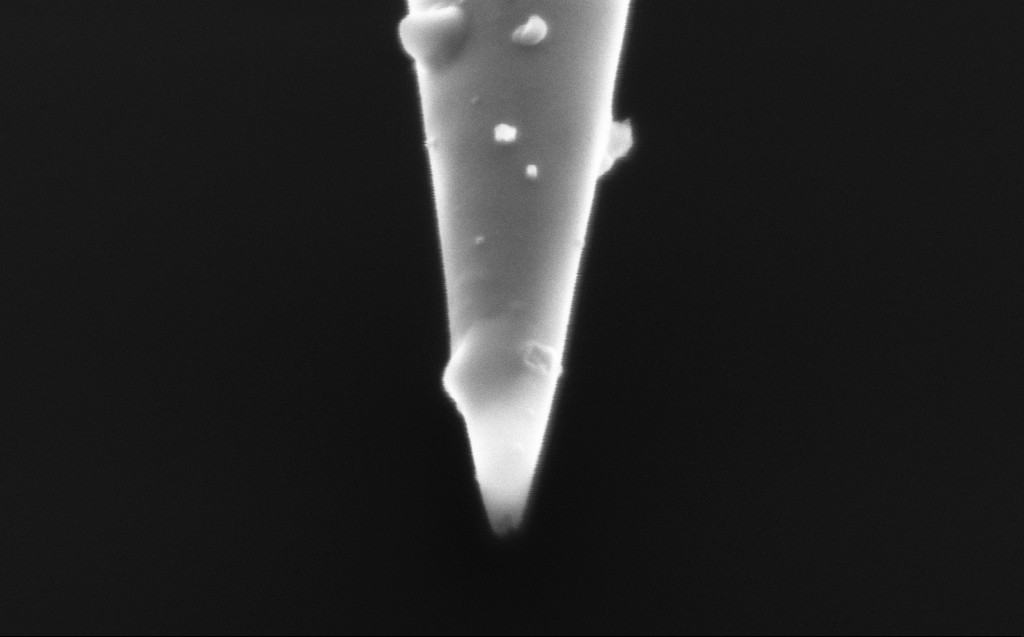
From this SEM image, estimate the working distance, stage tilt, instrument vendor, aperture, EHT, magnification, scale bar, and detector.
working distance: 2 mm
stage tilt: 45.1°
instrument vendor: Zeiss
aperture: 20 µm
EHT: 2.5 kV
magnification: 136.82 K X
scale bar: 200 nm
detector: InLens